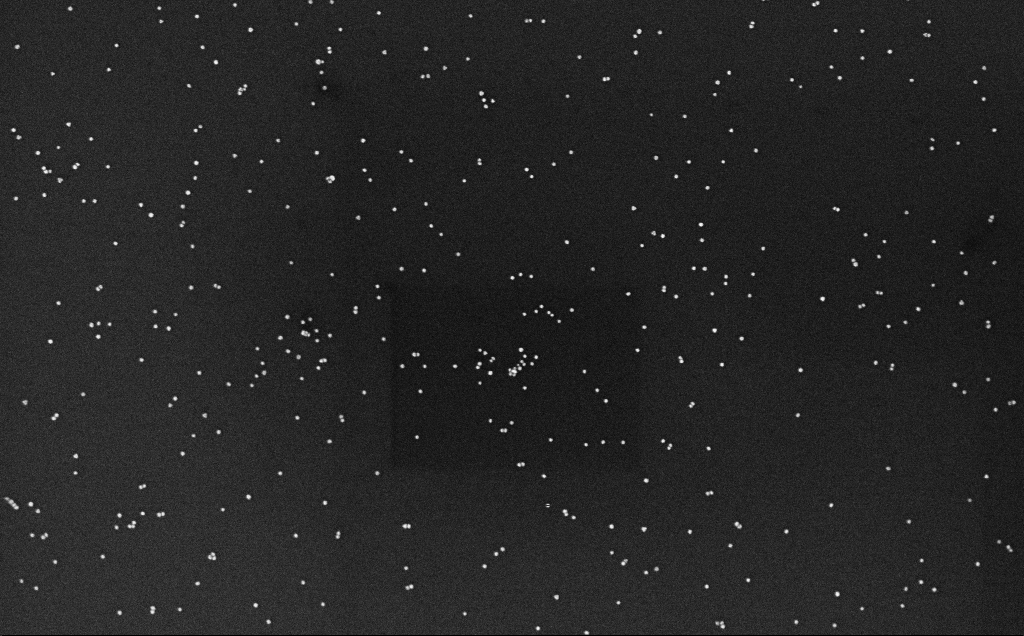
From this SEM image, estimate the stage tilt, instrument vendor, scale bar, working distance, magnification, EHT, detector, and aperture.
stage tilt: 0°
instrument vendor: Zeiss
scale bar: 200 nm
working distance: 3.2 mm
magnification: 100 K X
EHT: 10 kV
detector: InLens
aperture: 30 µm